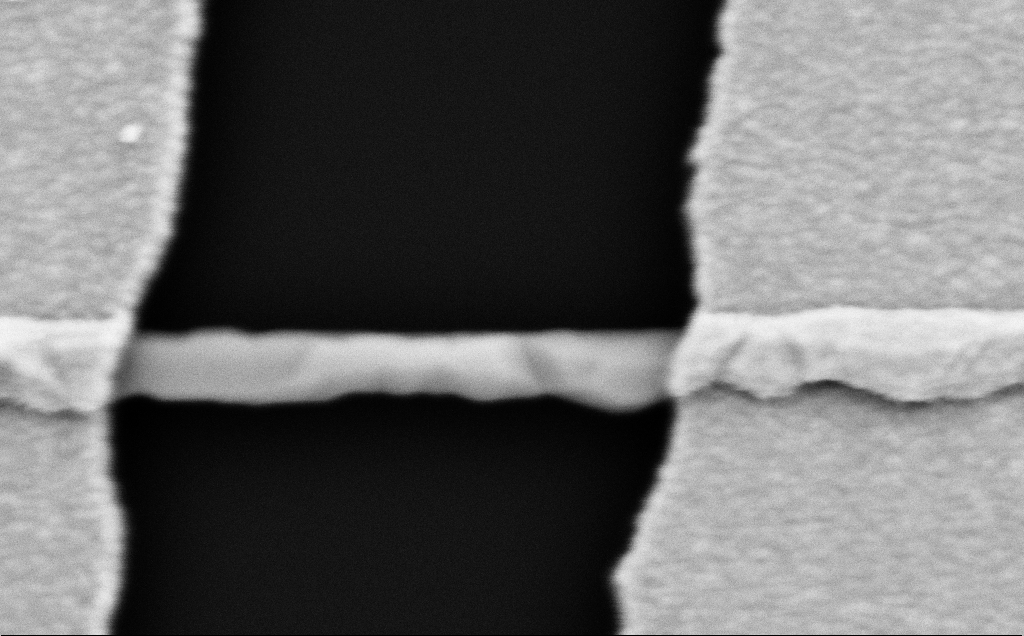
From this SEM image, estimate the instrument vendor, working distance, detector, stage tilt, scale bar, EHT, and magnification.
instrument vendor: Zeiss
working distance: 10 mm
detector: SE2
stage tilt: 0°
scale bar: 200 nm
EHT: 5 kV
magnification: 132.44 K X